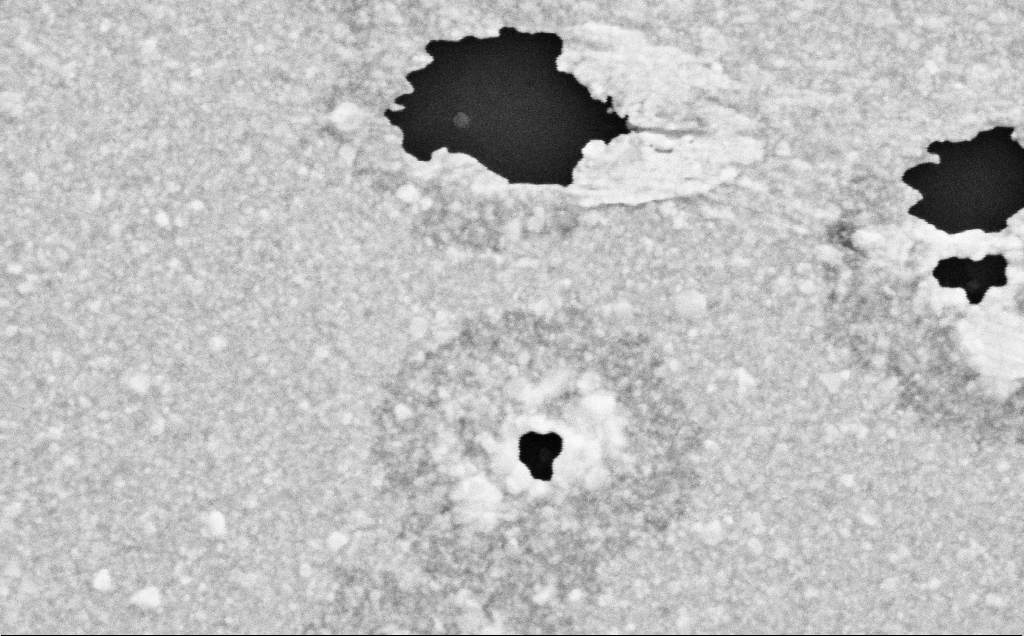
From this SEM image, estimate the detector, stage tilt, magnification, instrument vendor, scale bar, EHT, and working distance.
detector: SE2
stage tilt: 0°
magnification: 113.41 K X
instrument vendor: Zeiss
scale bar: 200 nm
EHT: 5 kV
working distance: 12 mm